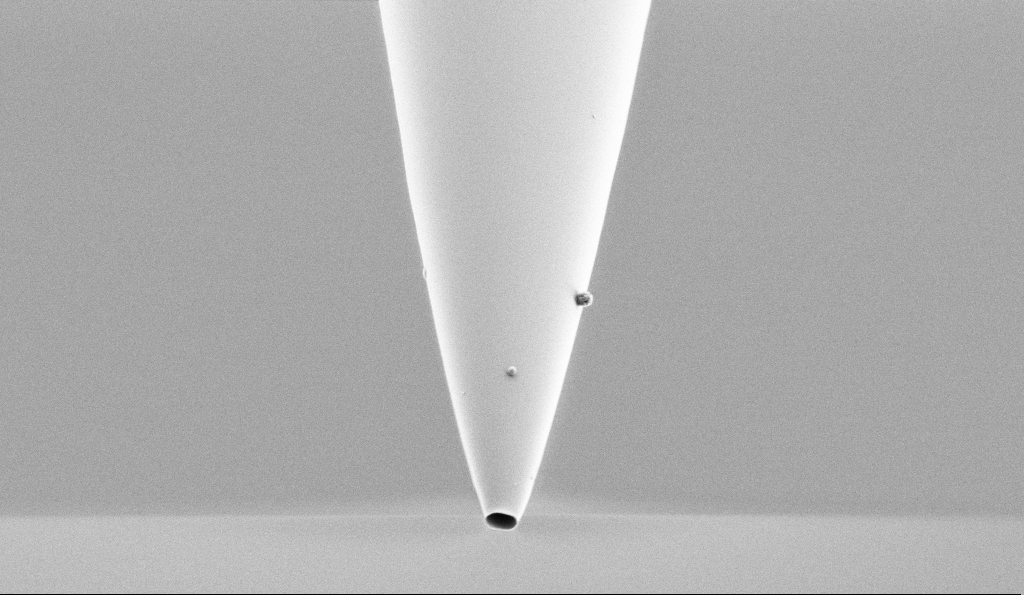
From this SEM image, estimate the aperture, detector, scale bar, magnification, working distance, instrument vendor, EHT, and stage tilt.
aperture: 30 µm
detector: SE2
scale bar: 1000 nm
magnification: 15 K X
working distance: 7.5 mm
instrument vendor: Zeiss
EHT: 1 kV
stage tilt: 45°